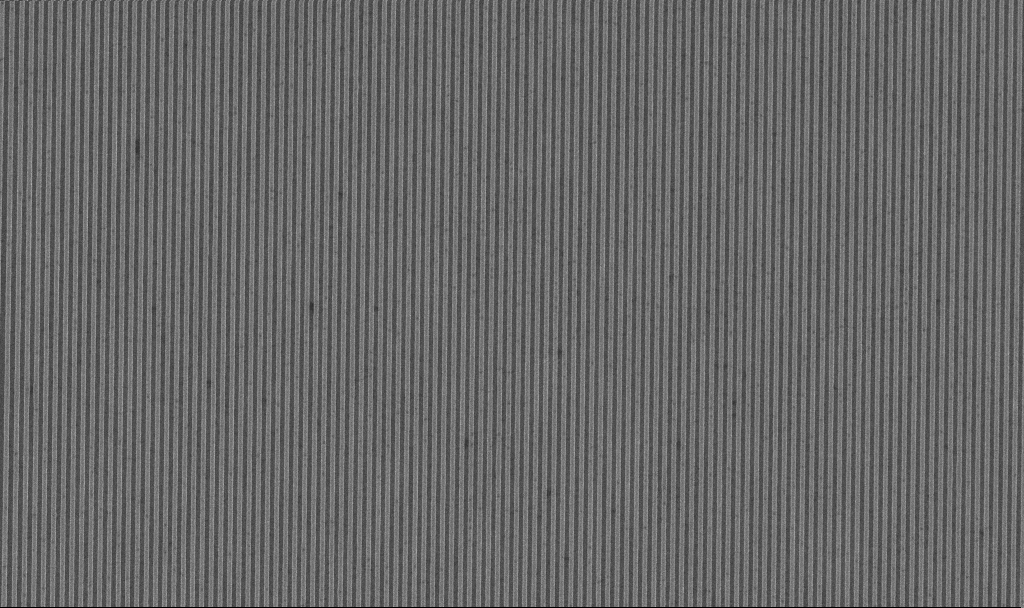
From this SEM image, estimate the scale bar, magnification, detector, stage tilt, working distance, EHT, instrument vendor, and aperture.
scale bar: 2000 nm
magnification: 8.32 K X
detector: InLens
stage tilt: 0°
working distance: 7.8 mm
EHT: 10 kV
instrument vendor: Zeiss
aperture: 30 µm